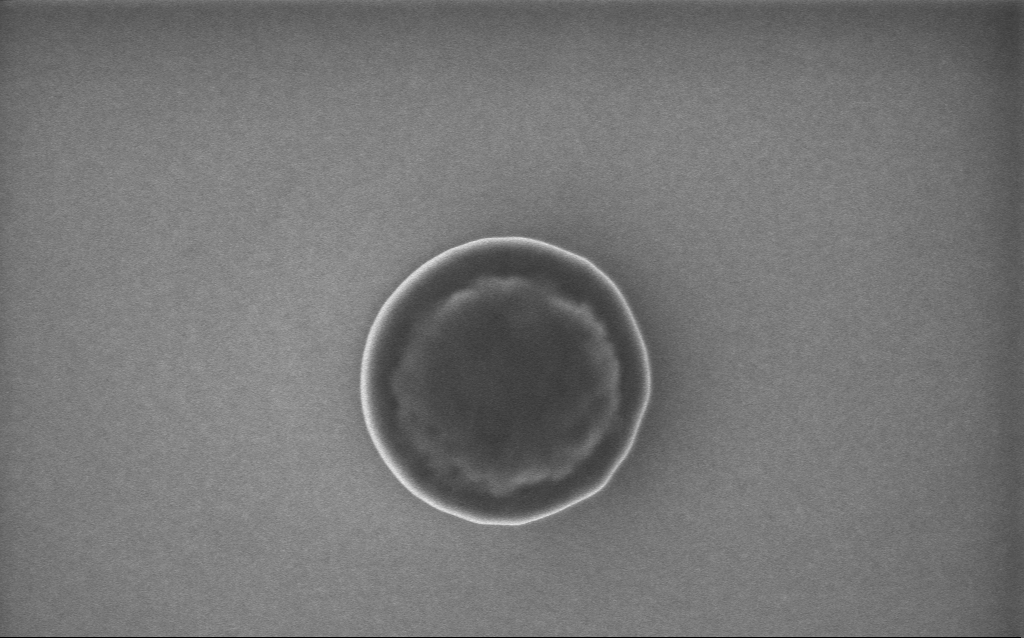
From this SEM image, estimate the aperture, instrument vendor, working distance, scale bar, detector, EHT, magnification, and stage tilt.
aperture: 30 µm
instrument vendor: Zeiss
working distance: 4 mm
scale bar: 1000 nm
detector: InLens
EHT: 5 kV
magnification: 64.05 K X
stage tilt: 0°